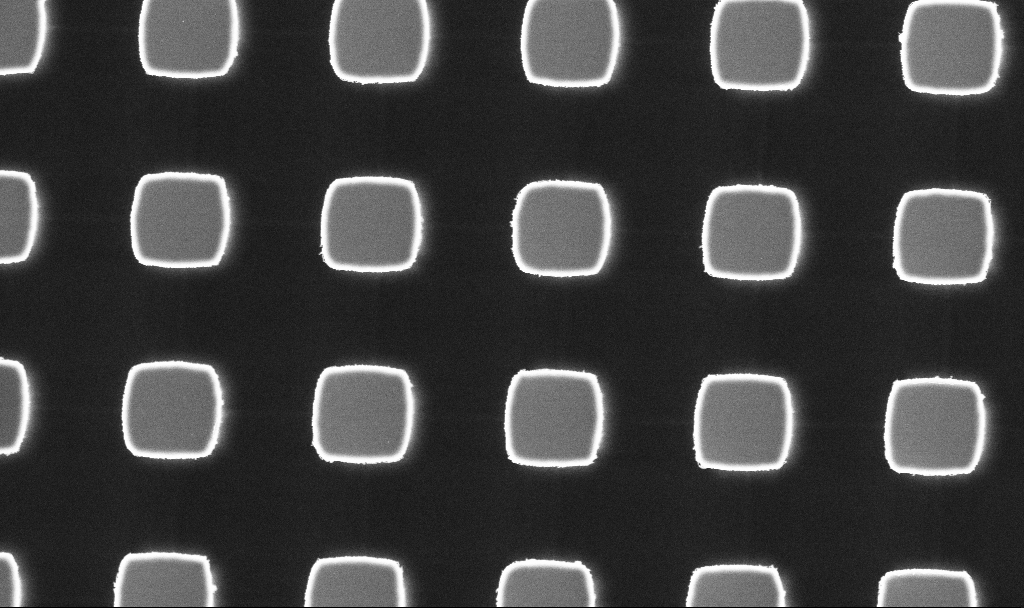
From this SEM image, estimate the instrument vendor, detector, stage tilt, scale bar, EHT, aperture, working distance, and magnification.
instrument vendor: Zeiss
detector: InLens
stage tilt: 0°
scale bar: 1000 nm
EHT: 3 kV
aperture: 30 µm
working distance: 2.9 mm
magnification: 17.66 K X